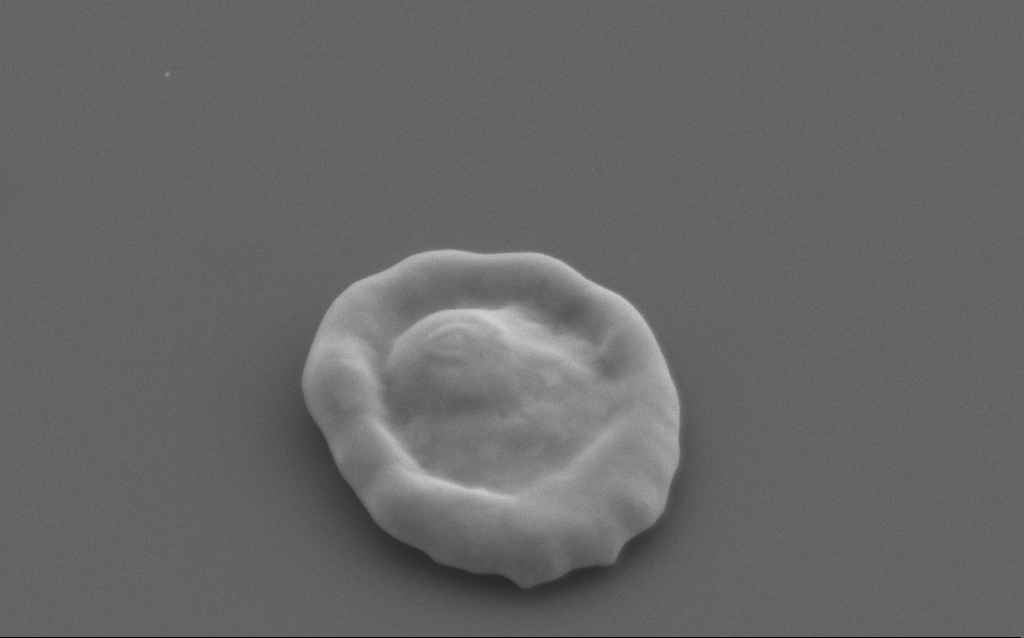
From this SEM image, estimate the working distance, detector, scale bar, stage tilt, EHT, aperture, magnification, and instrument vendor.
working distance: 5 mm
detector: SE2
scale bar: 1000 nm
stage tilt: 40°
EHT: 4 kV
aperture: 30 µm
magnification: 69.64 K X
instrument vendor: Zeiss